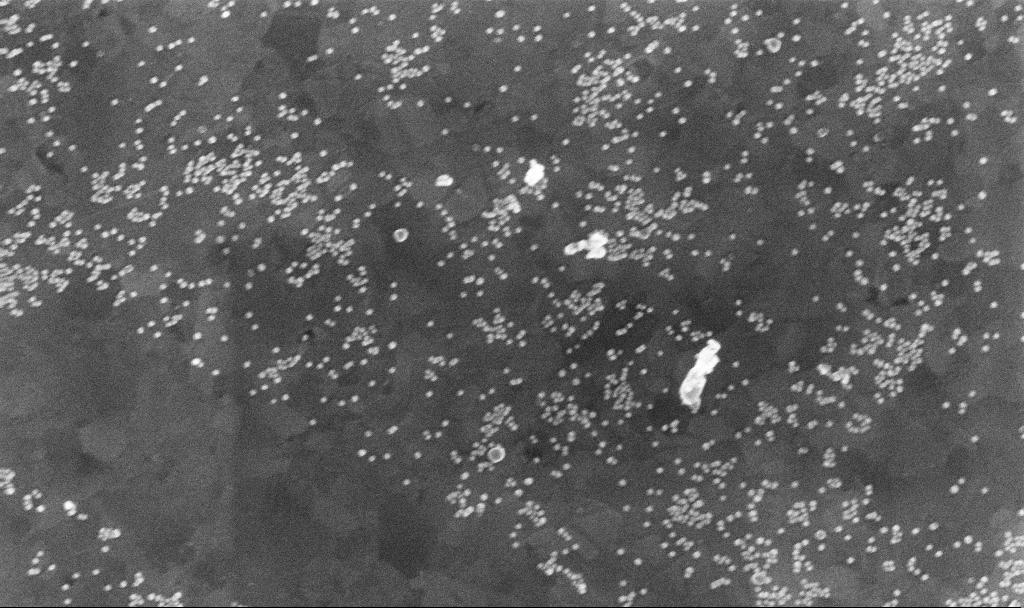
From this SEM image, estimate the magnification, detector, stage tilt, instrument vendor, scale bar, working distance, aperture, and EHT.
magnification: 100 K X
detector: InLens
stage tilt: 0°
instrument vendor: Zeiss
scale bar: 200 nm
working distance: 3.7 mm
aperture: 30 µm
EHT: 10 kV